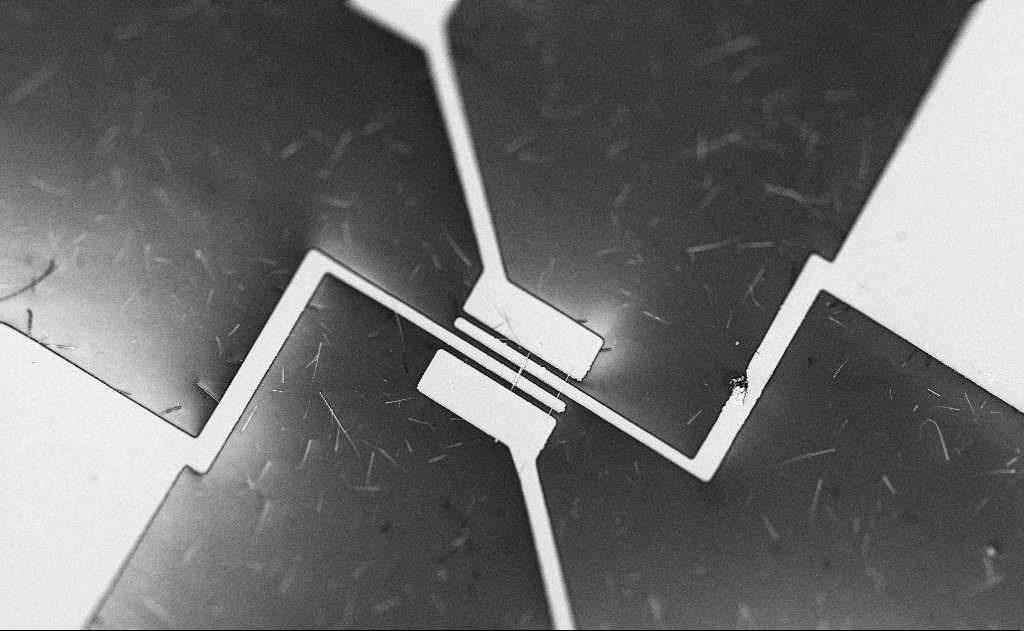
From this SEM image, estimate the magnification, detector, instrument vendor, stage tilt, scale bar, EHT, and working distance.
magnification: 1.66 K X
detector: SE2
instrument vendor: Zeiss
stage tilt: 0°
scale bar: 10000 nm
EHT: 5 kV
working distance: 20 mm